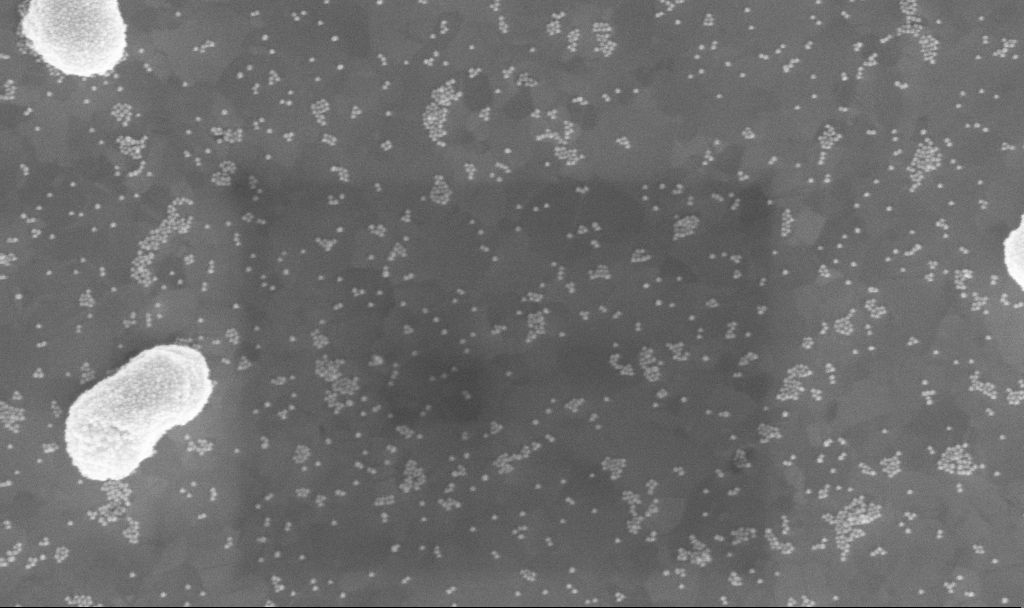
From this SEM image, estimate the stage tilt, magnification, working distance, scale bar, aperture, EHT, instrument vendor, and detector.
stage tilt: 0°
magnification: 100.62 K X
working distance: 3.9 mm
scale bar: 200 nm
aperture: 30 µm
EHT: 10 kV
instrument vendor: Zeiss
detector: InLens